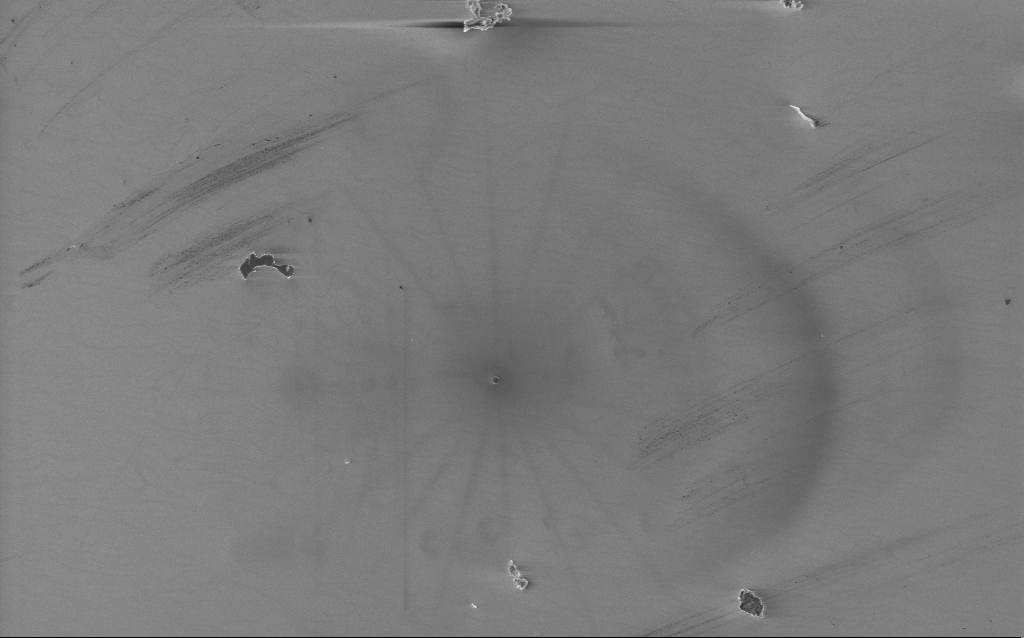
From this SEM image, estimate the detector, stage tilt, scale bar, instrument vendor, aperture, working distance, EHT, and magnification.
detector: InLens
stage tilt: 0°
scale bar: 10000 nm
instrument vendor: Zeiss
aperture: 30 µm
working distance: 4 mm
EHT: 5 kV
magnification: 2.65 K X